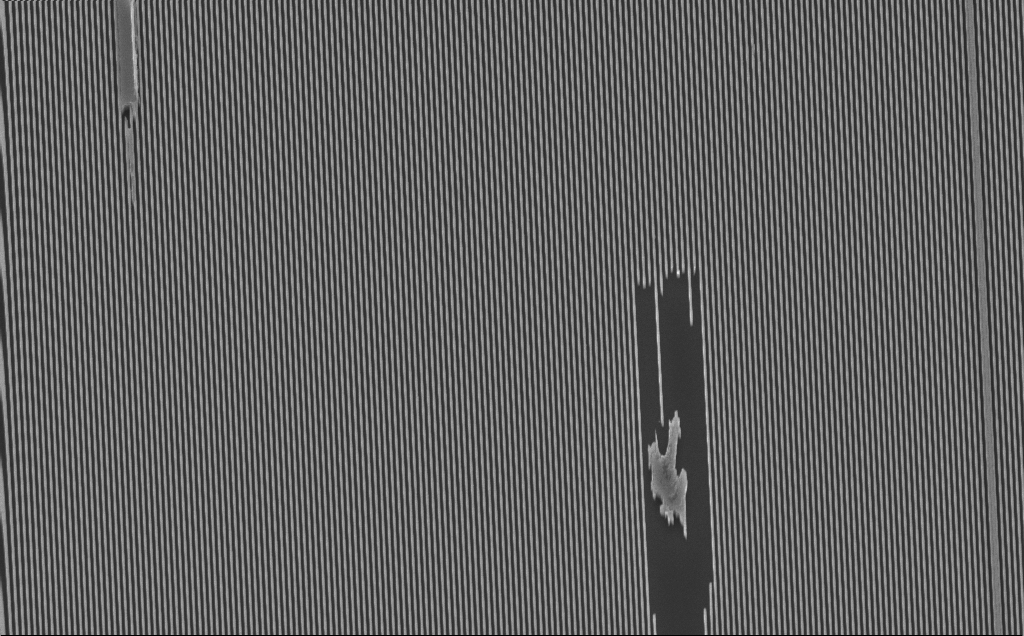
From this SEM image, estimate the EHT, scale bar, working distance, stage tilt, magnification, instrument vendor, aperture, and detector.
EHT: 10 kV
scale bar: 10000 nm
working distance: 4 mm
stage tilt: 25°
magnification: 4.2 K X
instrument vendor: Zeiss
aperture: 30 µm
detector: InLens